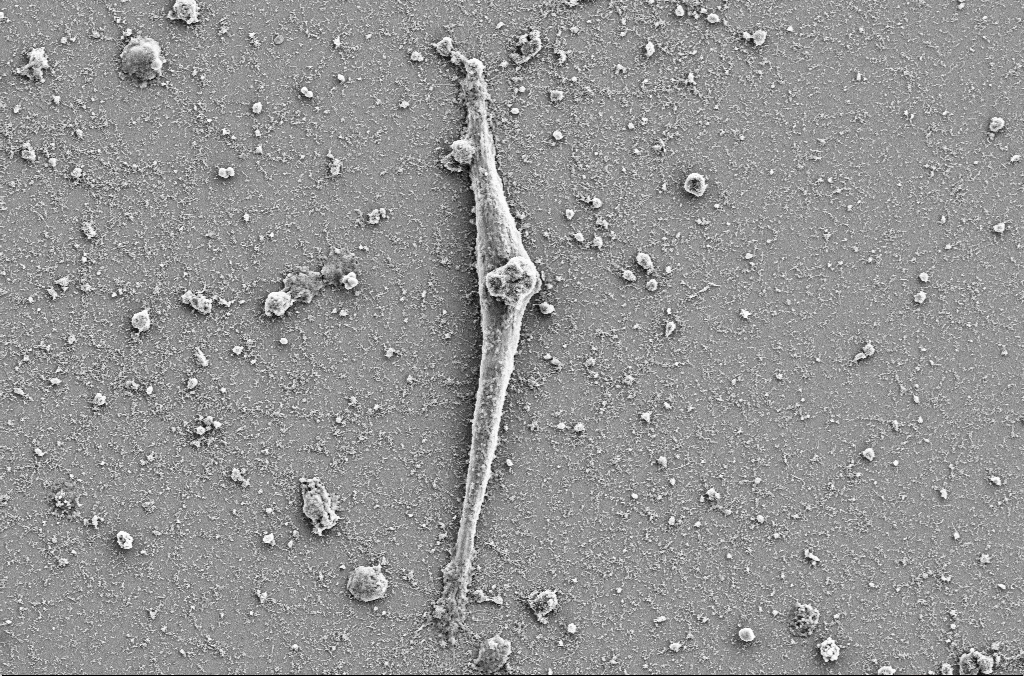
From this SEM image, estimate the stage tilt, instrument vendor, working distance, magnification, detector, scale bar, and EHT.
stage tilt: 0°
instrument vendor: Zeiss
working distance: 4 mm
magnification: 3 K X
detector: SE2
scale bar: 10000 nm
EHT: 5 kV